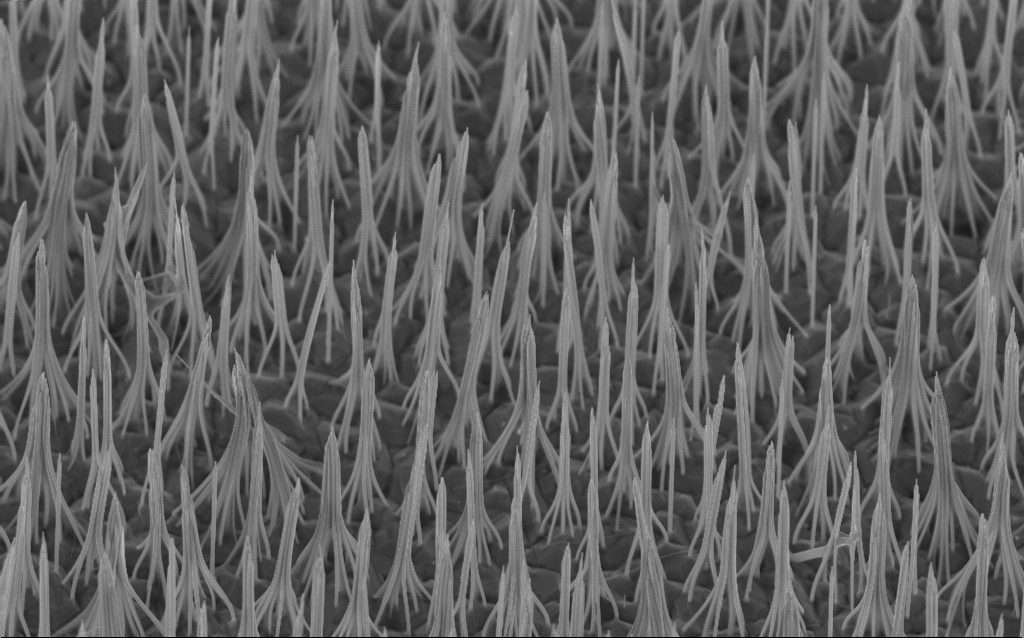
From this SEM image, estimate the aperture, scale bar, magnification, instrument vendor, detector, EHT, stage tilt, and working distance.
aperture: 30 µm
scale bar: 1000 nm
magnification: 17.41 K X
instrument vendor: Zeiss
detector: InLens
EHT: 5 kV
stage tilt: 40°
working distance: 6.3 mm